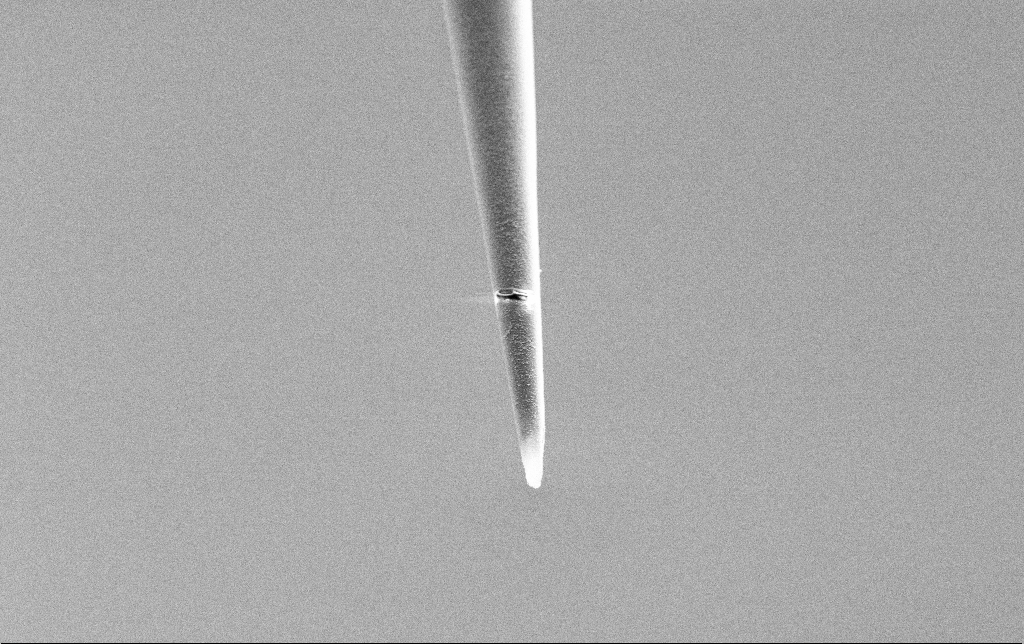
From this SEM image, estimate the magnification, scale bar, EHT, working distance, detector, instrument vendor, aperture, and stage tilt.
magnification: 5 K X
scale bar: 10000 nm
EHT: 3 kV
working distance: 7.5 mm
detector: SE2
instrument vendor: Zeiss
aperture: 30 µm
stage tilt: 45°